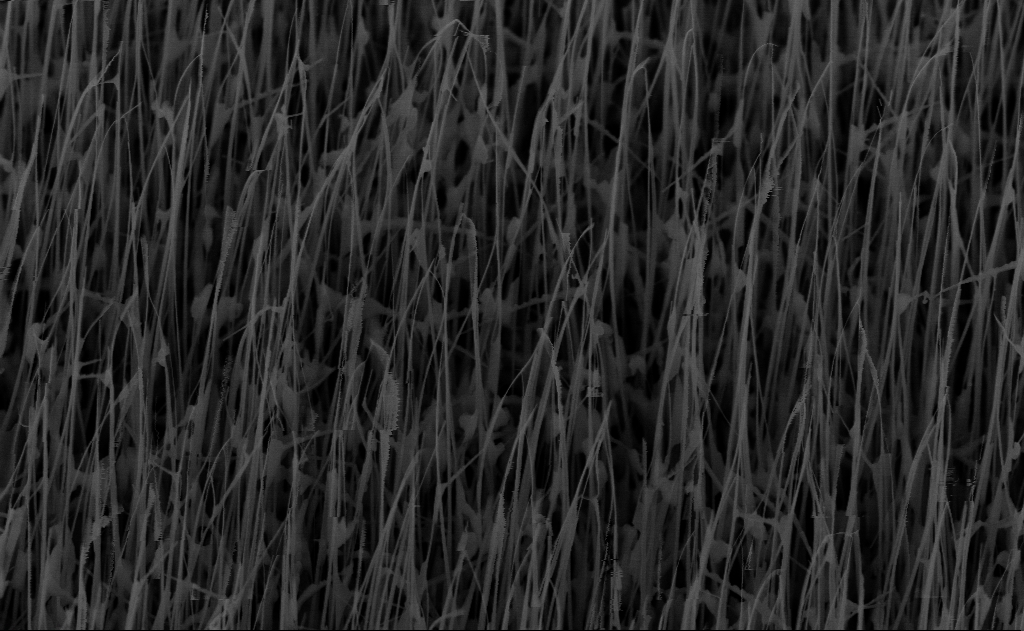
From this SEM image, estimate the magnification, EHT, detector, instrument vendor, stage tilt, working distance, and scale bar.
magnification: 40 K X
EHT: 10 kV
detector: InLens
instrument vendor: Zeiss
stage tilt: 45°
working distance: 7 mm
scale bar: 1000 nm